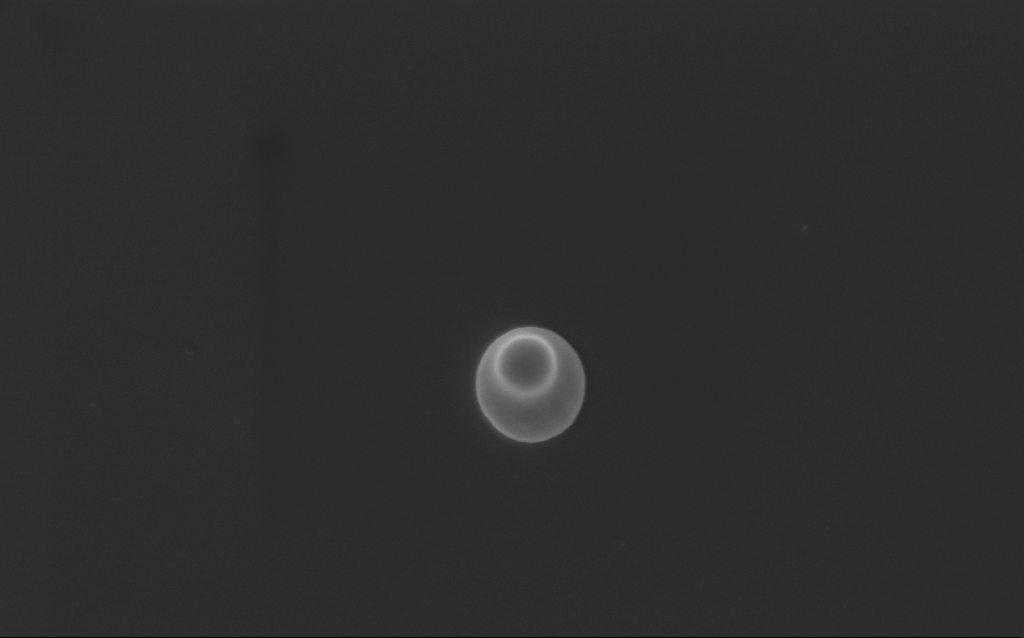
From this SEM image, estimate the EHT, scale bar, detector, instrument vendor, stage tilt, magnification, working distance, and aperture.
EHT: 3 kV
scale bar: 1000 nm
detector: InLens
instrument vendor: Zeiss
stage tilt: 0°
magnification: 44.45 K X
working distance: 4 mm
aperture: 30 µm